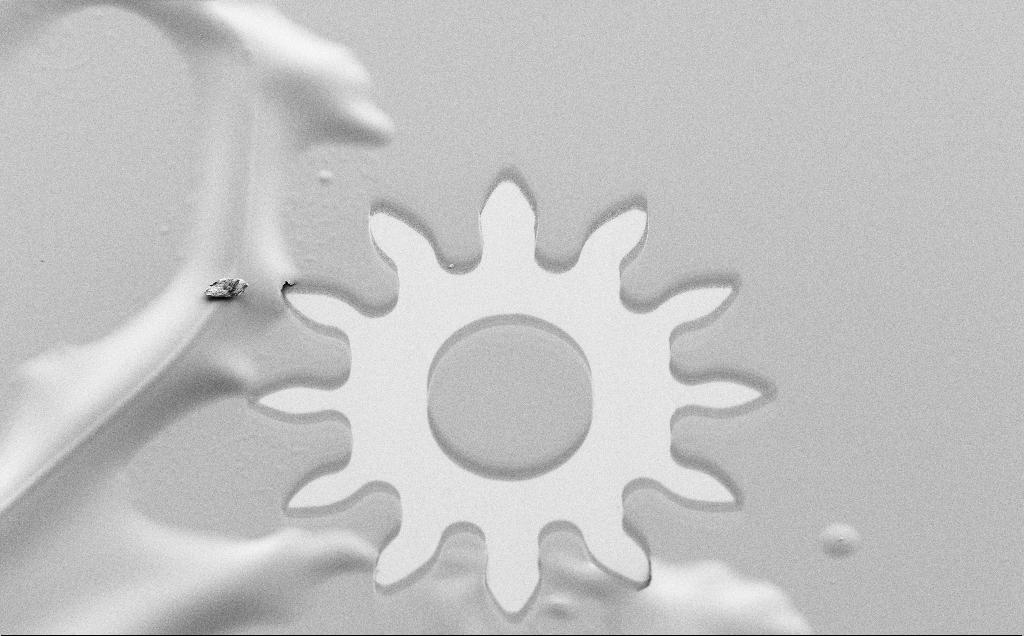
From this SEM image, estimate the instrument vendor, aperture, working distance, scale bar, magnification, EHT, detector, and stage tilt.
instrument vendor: Zeiss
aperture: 30 µm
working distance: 6 mm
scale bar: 100000 nm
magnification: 0.438 K X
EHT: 1.5 kV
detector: SE2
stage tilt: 30°